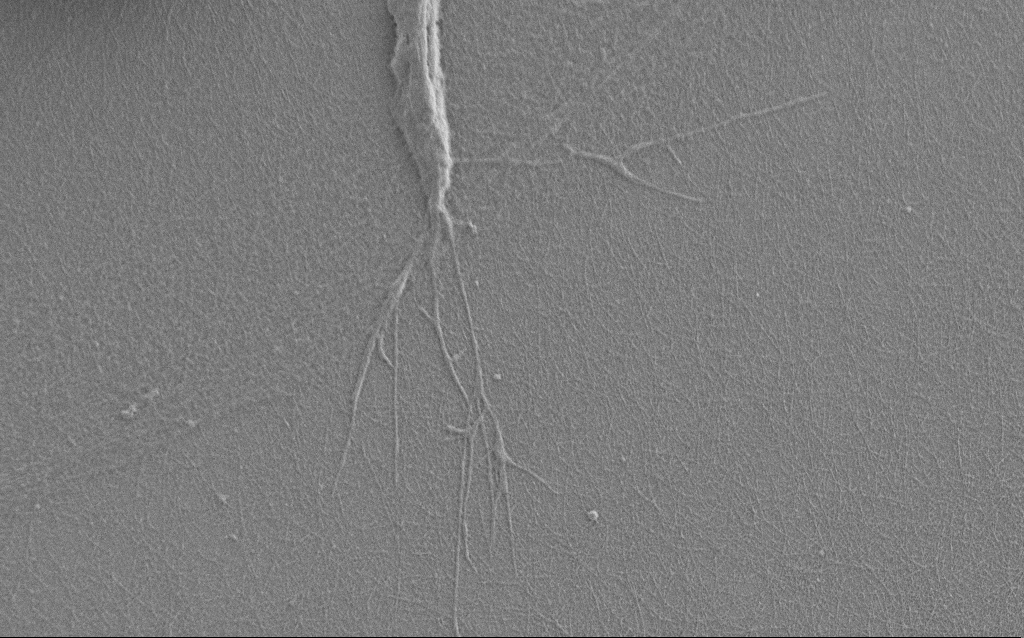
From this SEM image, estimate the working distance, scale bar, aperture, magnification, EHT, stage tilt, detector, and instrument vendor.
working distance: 6 mm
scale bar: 2000 nm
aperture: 30 µm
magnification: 7.5 K X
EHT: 1 kV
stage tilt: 0°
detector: SE2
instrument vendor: Zeiss